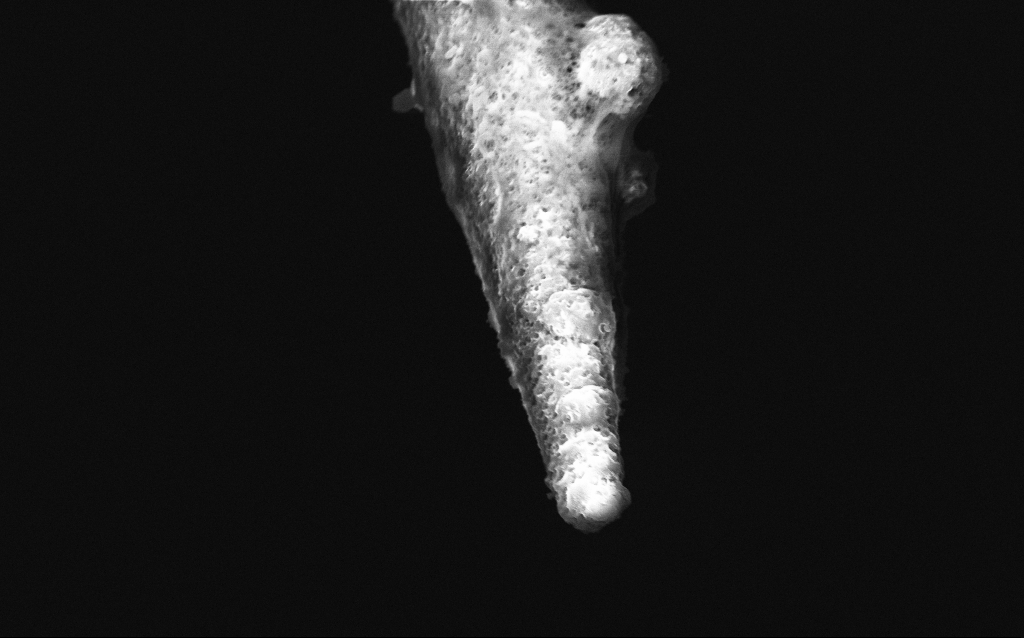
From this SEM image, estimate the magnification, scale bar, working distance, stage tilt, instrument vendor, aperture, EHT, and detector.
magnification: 25 K X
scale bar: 1000 nm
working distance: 5.8 mm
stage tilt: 43.9°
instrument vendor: Zeiss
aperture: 30 µm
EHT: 5 kV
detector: InLens